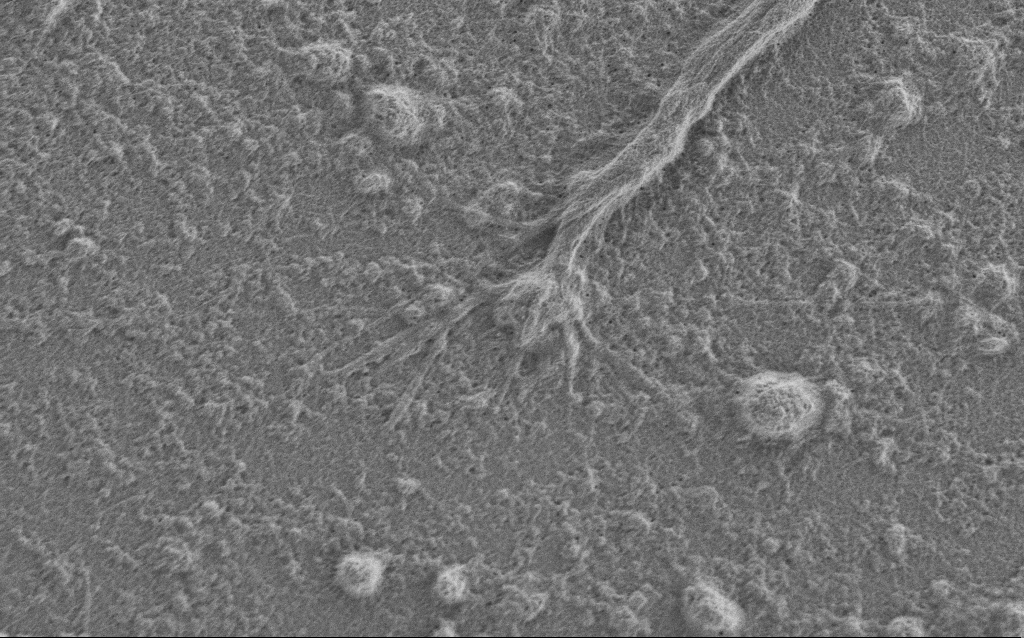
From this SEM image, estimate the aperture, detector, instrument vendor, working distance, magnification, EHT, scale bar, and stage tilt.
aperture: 30 µm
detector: SE2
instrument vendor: Zeiss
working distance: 6 mm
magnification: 10 K X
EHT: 1 kV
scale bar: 2000 nm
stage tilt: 0°